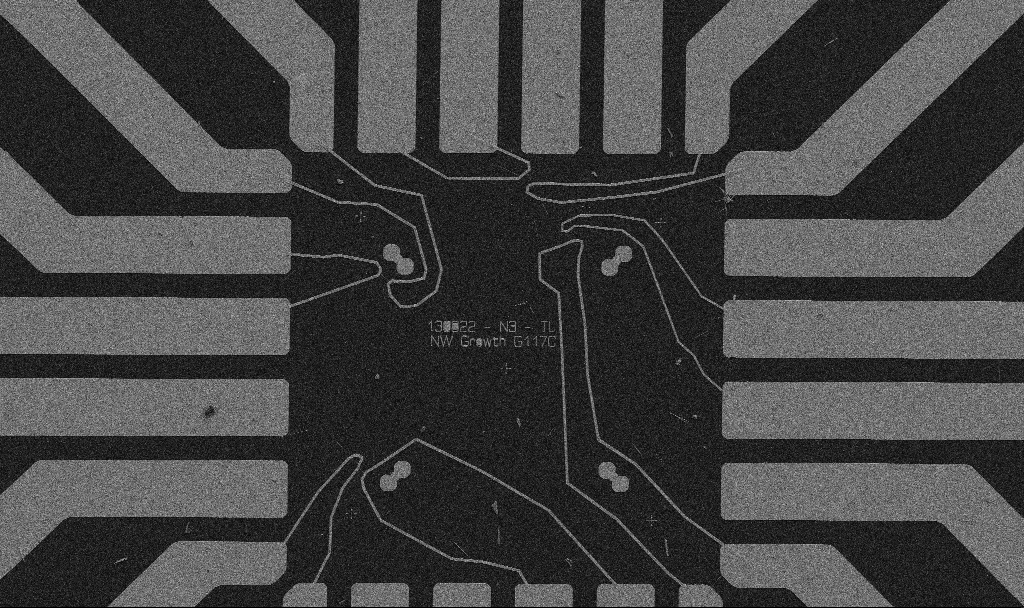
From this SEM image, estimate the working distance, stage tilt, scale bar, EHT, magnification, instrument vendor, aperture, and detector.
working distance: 10.7 mm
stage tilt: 0°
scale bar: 20000 nm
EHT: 5 kV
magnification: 1 K X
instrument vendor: Zeiss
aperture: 30 µm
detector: SE2